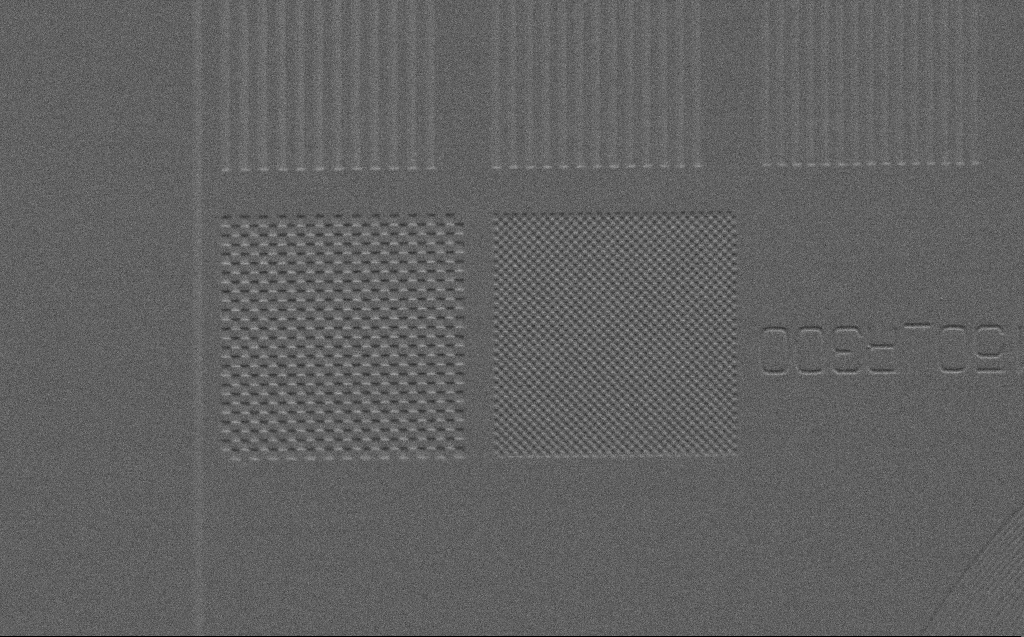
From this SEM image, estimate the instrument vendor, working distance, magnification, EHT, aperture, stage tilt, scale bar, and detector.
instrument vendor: Zeiss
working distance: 4 mm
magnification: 3.5 K X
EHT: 2 kV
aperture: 30 µm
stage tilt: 45°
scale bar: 10000 nm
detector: SE2